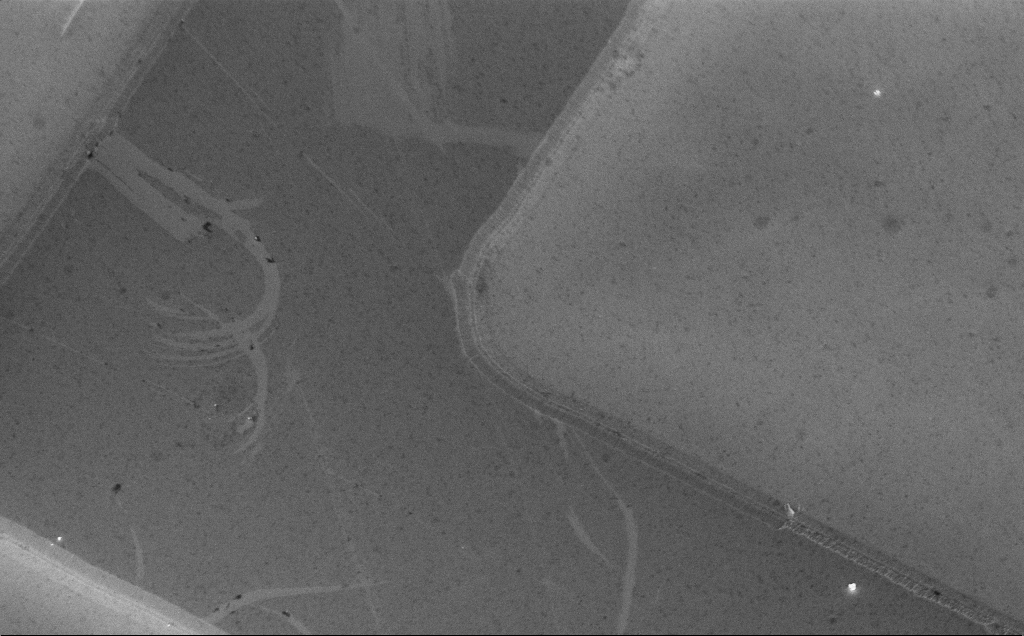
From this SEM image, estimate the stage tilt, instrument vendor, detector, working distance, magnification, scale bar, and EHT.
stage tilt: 30°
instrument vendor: Zeiss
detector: InLens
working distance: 8 mm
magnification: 8.46 K X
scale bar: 2000 nm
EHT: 5 kV